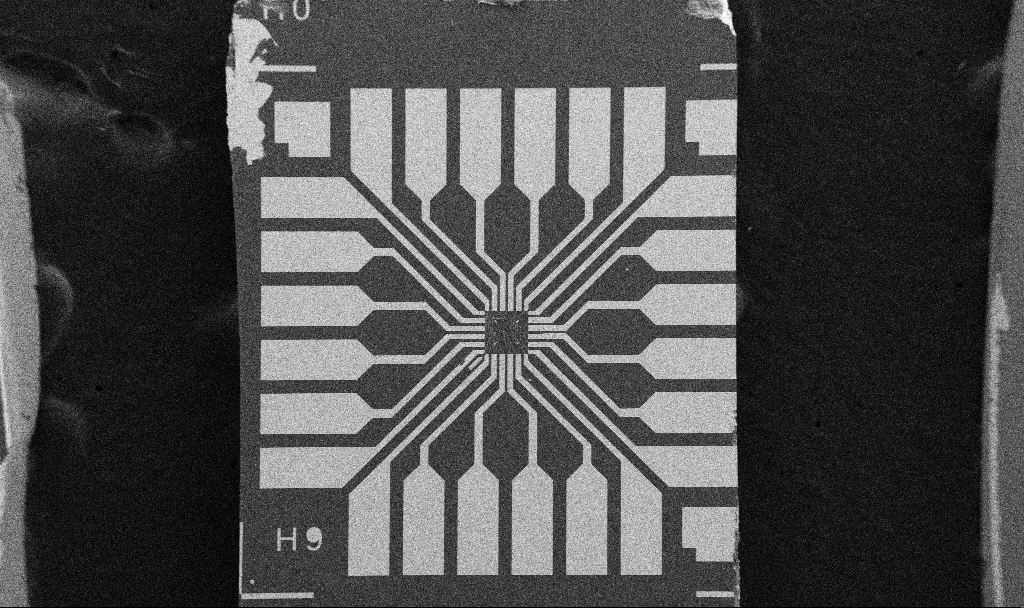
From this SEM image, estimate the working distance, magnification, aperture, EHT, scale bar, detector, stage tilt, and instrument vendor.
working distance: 10.7 mm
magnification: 0.1 K X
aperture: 30 µm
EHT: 5 kV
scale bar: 200000 nm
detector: SE2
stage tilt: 0°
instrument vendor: Zeiss